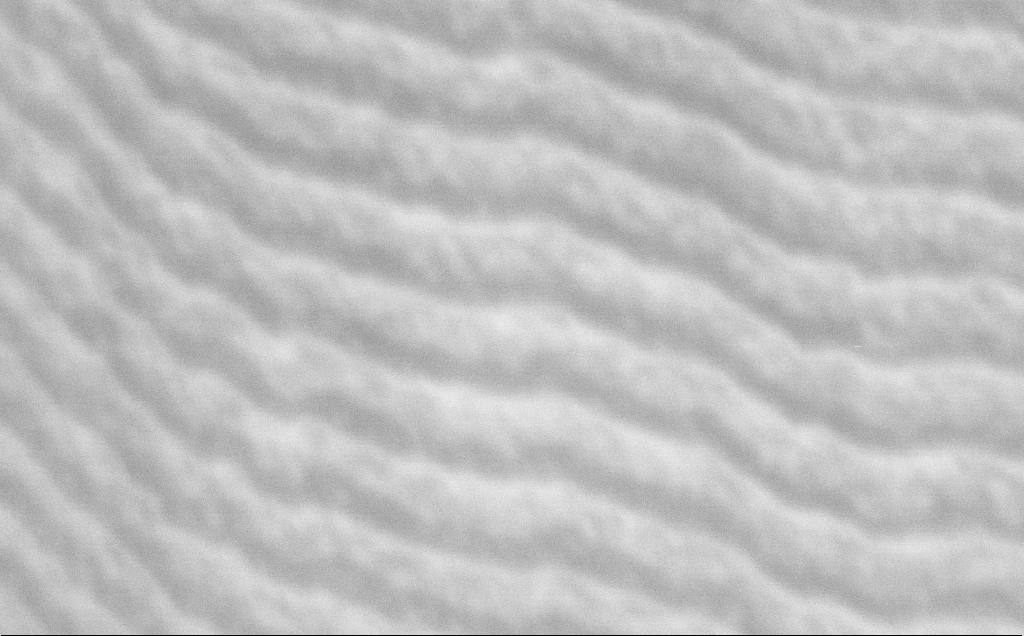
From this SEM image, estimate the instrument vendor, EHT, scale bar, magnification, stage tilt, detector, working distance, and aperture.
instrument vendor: Zeiss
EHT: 1.2 kV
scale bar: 200 nm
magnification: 89.28 K X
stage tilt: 45°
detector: InLens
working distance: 8 mm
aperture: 30 µm